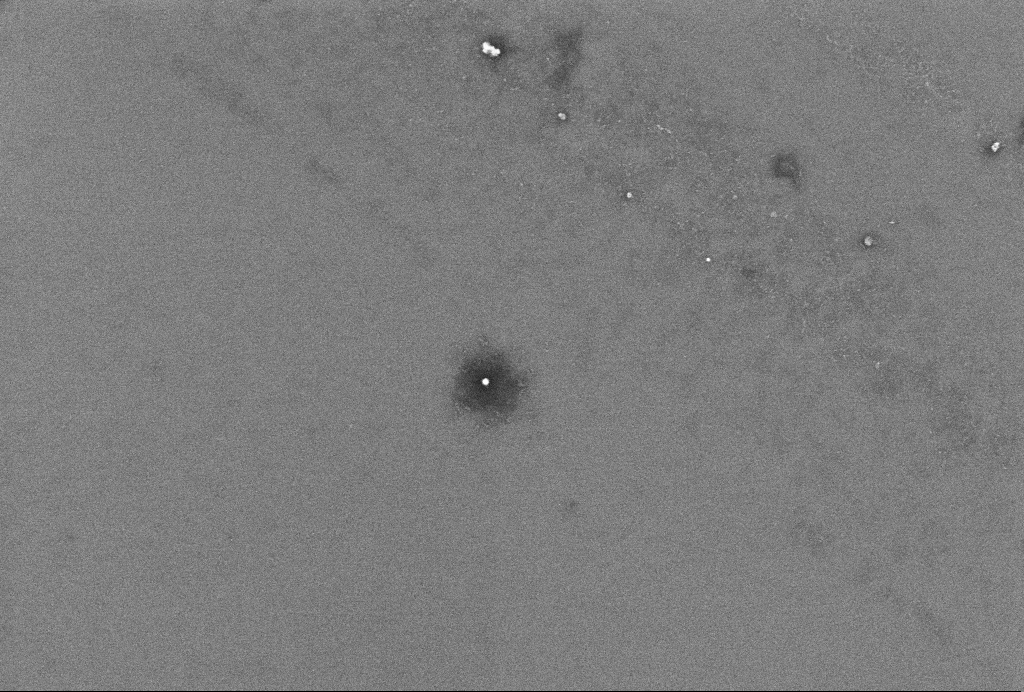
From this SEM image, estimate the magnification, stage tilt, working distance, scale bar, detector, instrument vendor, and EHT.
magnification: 86.19 K X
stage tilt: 0°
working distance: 3.3 mm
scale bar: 200 nm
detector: InLens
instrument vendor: Zeiss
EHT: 2 kV